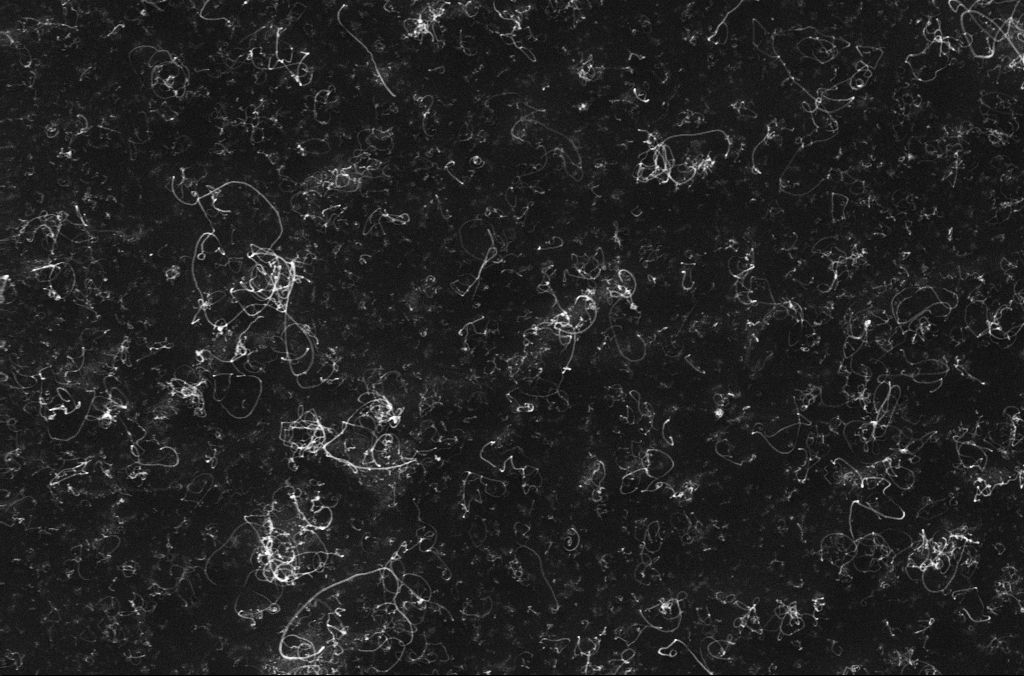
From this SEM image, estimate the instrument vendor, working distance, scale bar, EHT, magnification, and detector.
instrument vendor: Zeiss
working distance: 3.3 mm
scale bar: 2000 nm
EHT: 10 kV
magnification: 10 K X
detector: InLens